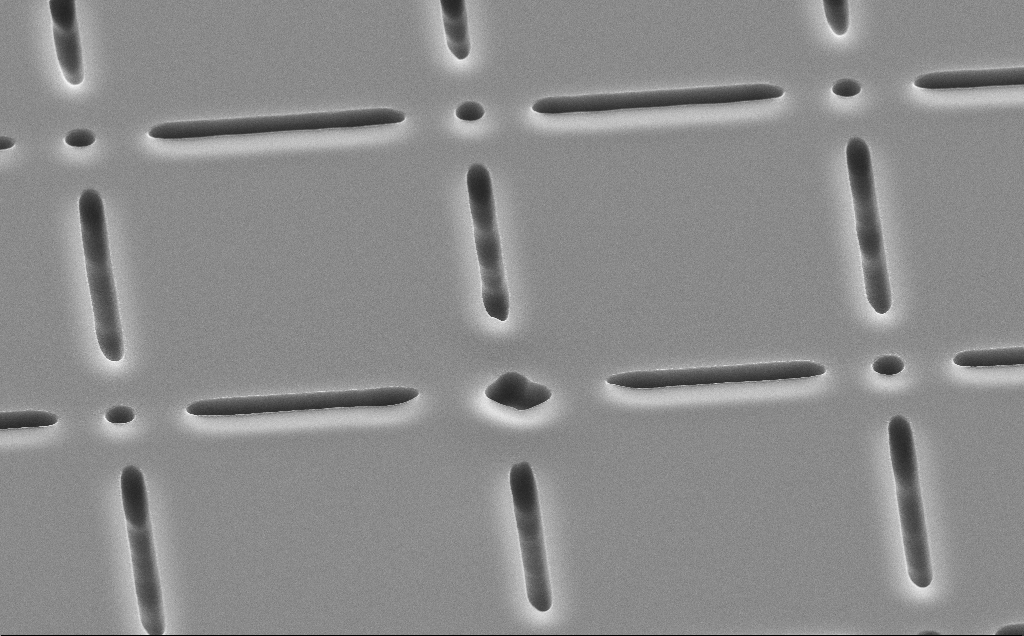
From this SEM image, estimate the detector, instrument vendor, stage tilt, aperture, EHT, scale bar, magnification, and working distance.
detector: SE2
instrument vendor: Zeiss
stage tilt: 45°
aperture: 30 µm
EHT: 10 kV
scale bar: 10000 nm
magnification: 7 K X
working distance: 11 mm